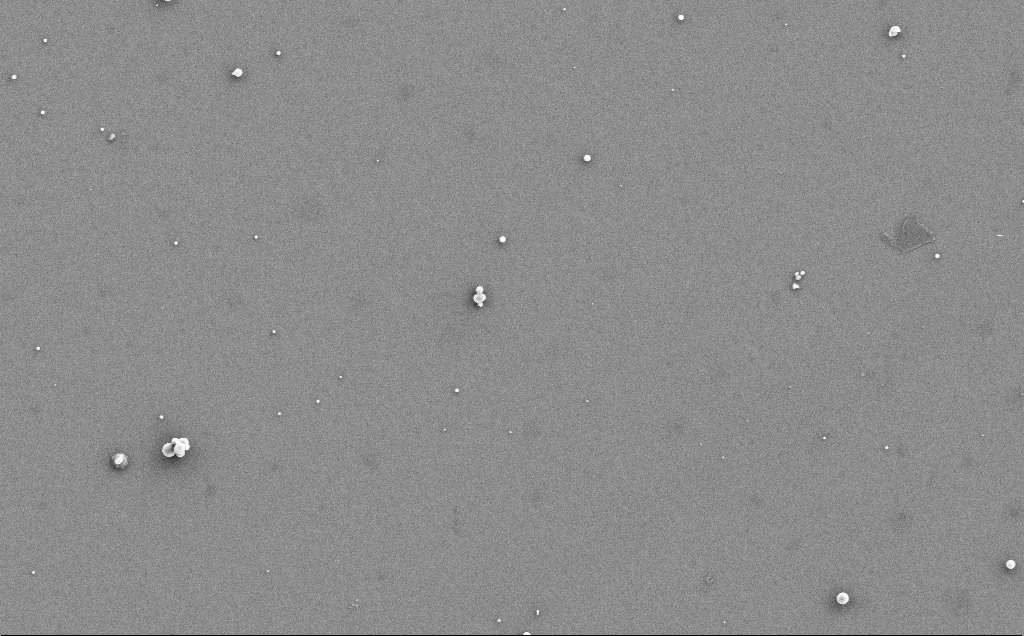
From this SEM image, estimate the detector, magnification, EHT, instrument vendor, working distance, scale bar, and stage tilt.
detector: SE2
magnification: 4.01 K X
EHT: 5 kV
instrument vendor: Zeiss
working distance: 5 mm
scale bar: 10000 nm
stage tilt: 0°